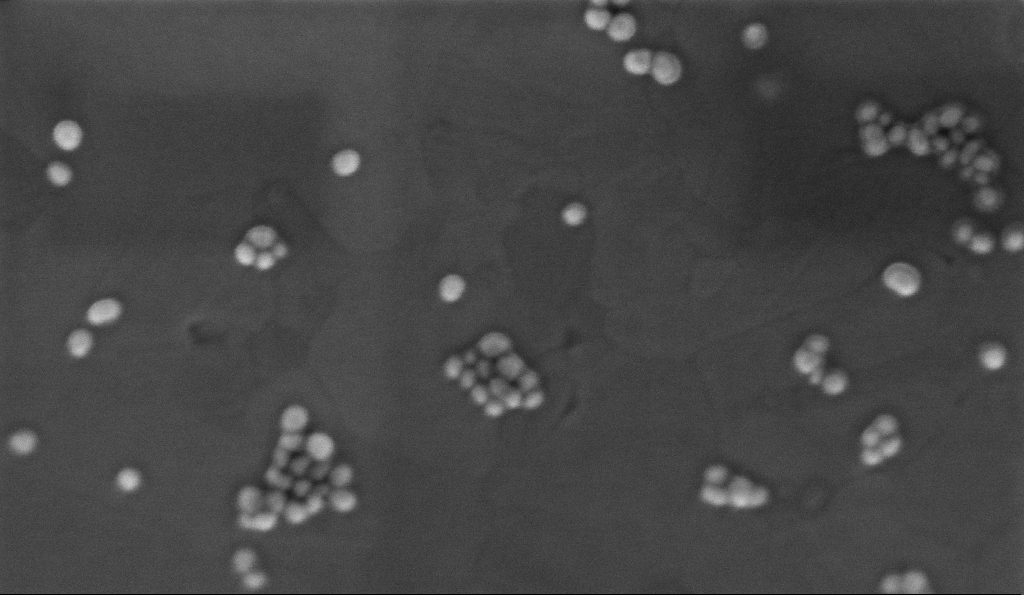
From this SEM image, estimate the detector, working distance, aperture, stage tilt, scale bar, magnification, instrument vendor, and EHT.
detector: InLens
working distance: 3.3 mm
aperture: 30 µm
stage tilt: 0°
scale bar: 200 nm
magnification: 300 K X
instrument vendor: Zeiss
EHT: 2 kV